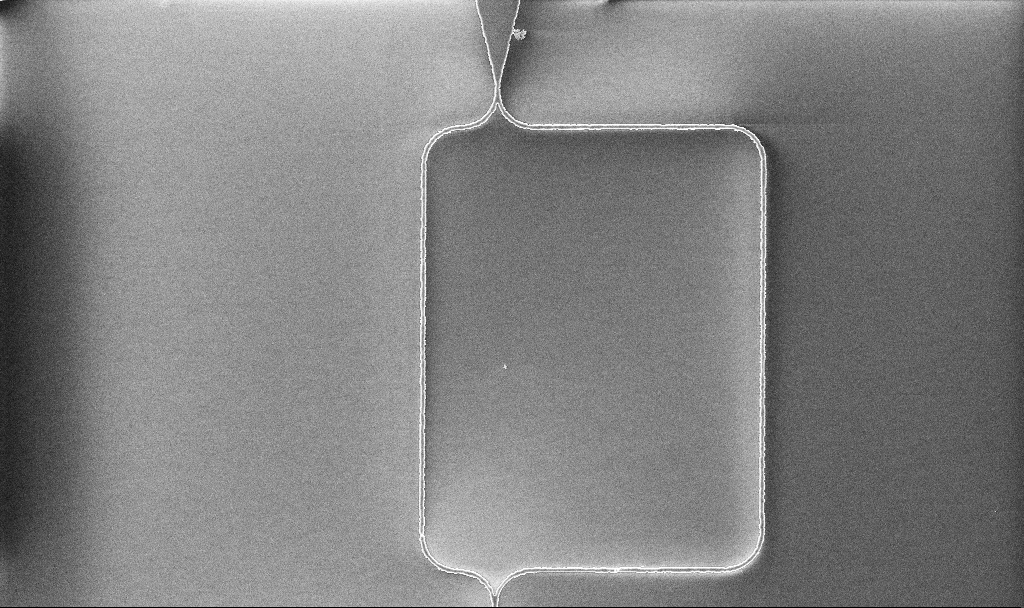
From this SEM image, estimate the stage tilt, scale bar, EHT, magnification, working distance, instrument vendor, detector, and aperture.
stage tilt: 0°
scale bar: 10000 nm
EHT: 5 kV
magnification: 2.86 K X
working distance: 10.1 mm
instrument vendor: Zeiss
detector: InLens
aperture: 30 µm